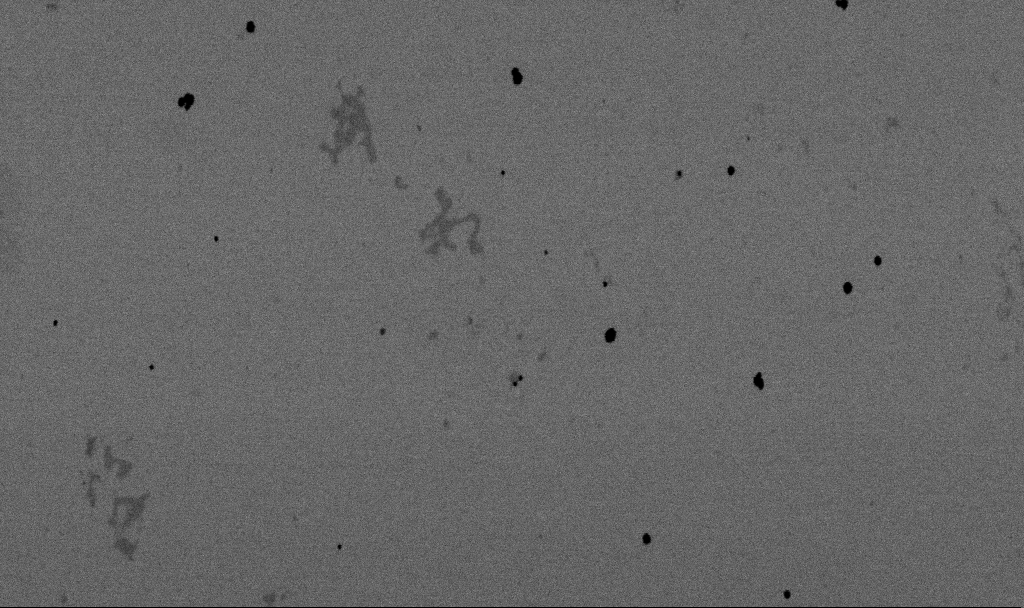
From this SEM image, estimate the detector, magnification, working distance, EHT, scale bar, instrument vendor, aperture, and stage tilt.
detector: SE2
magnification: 55 K X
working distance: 6.6 mm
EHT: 2 kV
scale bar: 1000 nm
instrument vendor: Zeiss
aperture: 30 µm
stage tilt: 0°